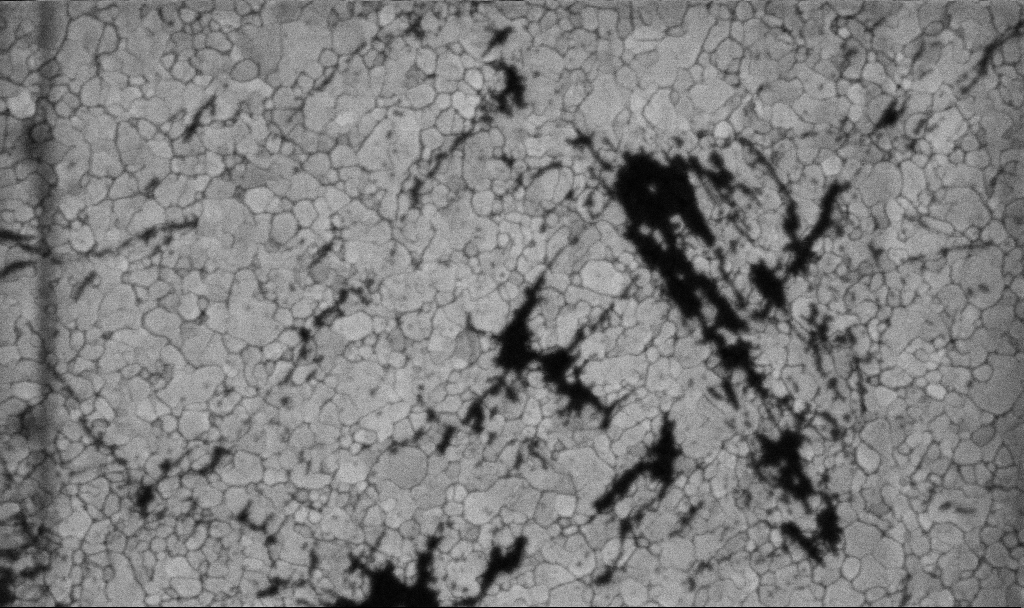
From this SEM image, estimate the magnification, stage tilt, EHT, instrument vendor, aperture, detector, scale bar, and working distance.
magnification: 100.15 K X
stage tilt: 0°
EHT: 5 kV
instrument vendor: Zeiss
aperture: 30 µm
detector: InLens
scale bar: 200 nm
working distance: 3.1 mm